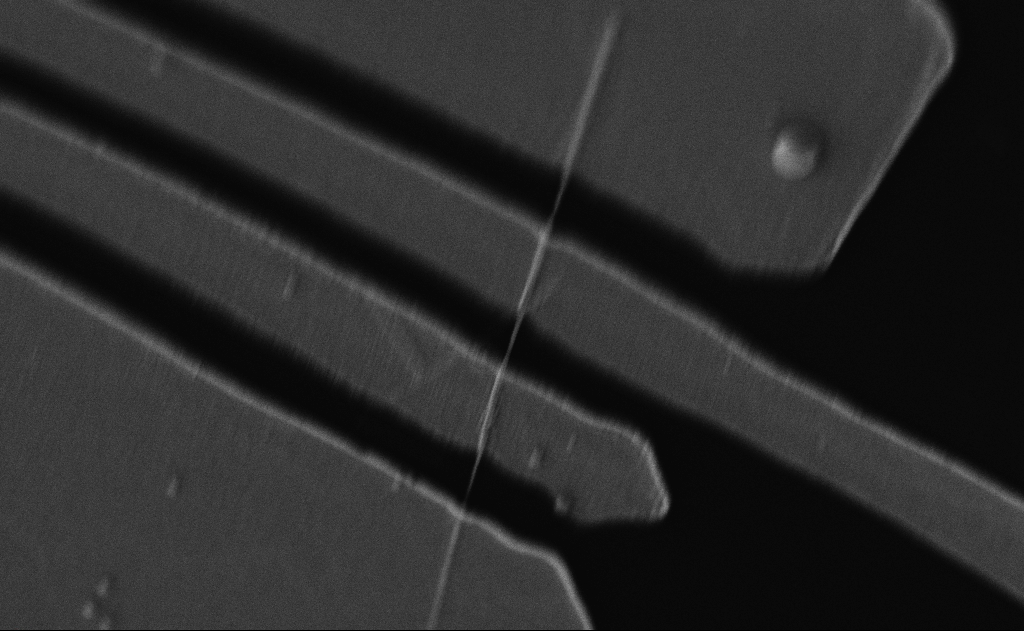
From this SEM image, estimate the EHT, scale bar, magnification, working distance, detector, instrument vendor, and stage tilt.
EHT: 5 kV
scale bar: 2000 nm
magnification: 13.93 K X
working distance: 22 mm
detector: InLens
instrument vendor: Zeiss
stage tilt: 0°